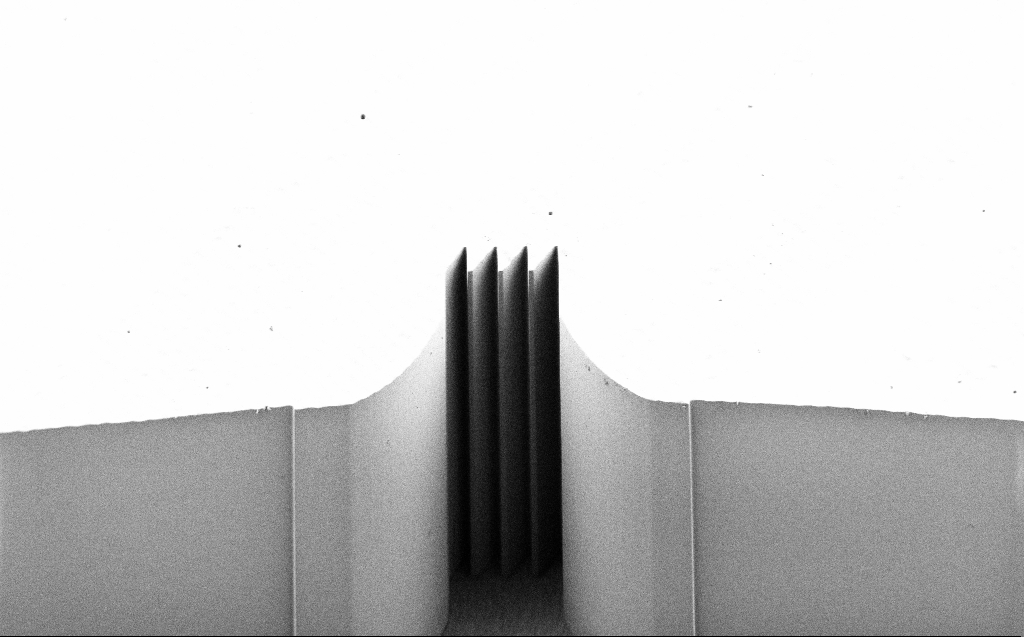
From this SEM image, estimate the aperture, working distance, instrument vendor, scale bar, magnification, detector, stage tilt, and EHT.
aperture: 30 µm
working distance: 7 mm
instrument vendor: Zeiss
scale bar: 20000 nm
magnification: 0.971 K X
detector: SE2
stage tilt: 45°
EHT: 1 kV